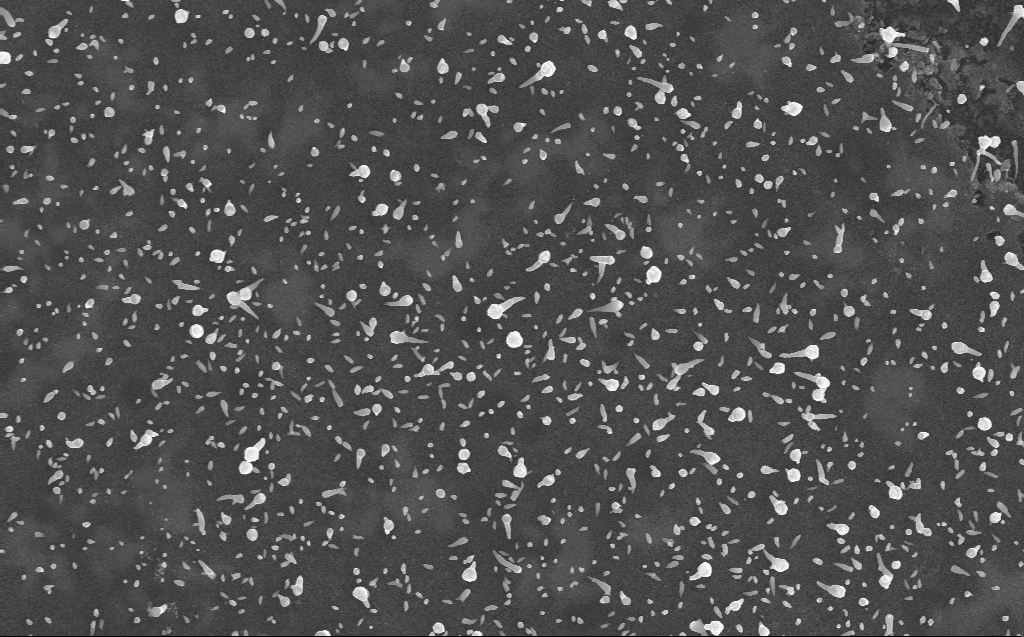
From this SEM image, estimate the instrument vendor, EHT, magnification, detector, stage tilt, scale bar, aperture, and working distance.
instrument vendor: Zeiss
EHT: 10 kV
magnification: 17.94 K X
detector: InLens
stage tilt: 0°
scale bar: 1000 nm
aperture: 30 µm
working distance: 3 mm